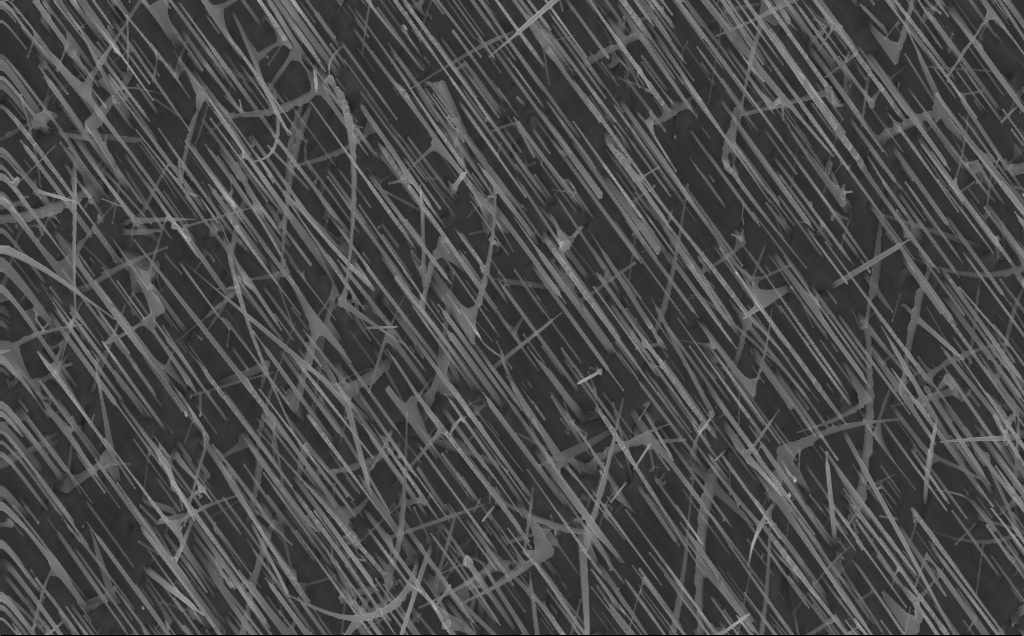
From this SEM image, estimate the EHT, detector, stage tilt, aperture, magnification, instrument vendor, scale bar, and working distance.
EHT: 10 kV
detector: InLens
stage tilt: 0°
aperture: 30 µm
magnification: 20 K X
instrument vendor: Zeiss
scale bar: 2000 nm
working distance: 4 mm